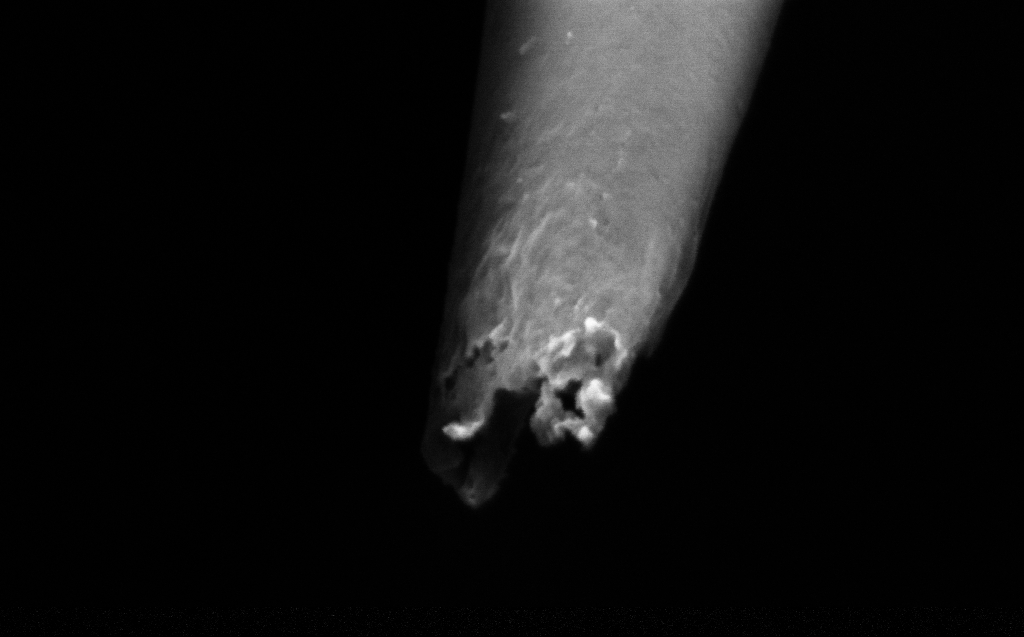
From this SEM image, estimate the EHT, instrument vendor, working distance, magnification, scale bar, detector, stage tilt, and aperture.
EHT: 2 kV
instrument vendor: Zeiss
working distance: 4 mm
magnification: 250 K X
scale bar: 200 nm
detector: InLens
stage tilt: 45°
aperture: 30 µm